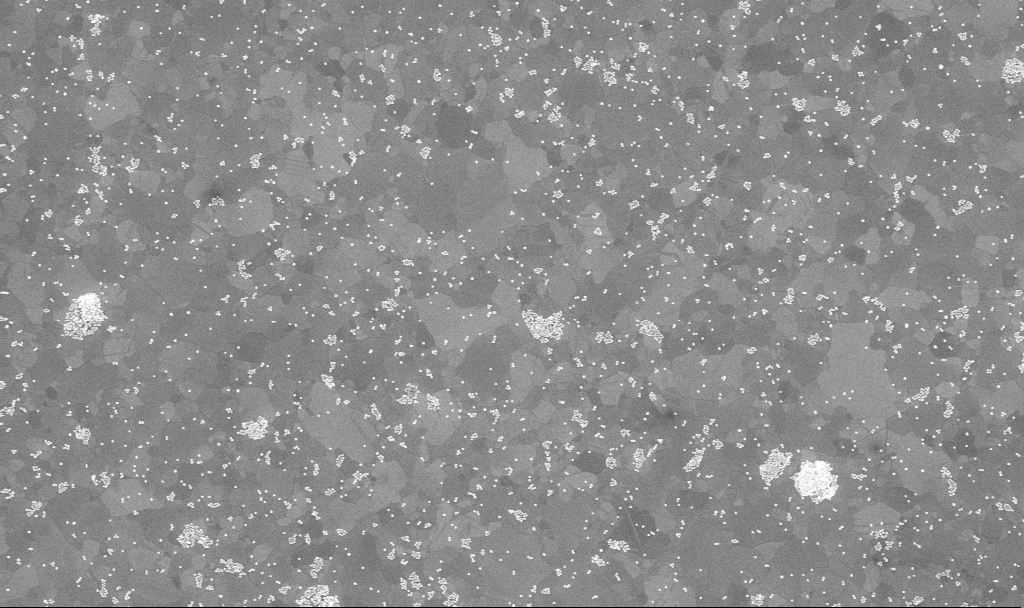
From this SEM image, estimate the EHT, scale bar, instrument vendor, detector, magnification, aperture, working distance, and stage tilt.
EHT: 10 kV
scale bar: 1000 nm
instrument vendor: Zeiss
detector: InLens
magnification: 50 K X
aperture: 30 µm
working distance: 3.8 mm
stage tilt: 0°